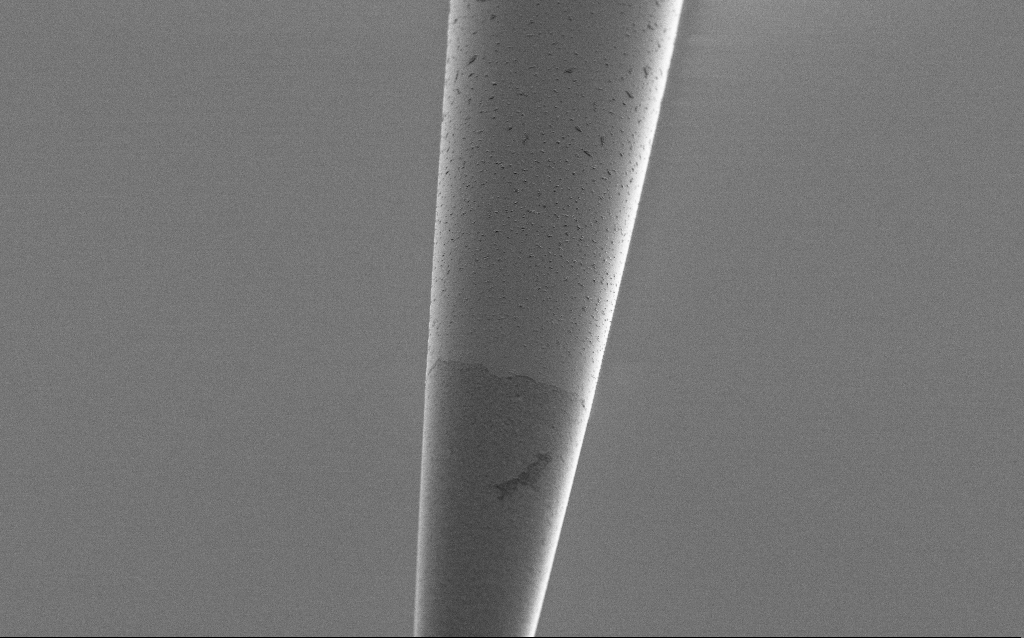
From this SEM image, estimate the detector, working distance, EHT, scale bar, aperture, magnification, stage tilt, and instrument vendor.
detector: SE2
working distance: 6 mm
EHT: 1 kV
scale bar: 10000 nm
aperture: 30 µm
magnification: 5 K X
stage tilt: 45°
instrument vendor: Zeiss